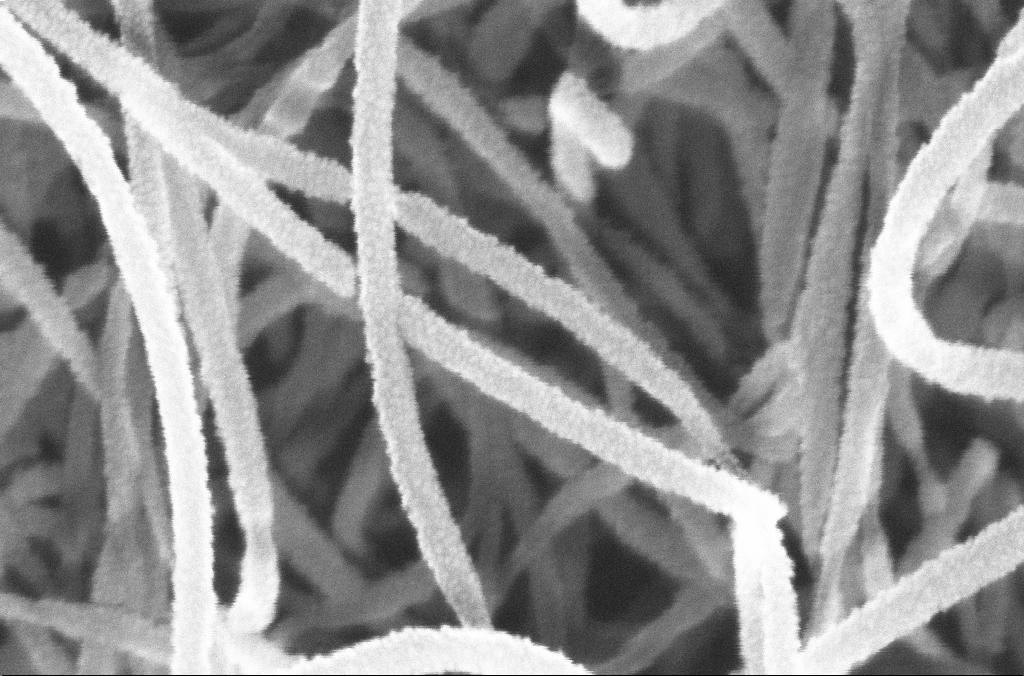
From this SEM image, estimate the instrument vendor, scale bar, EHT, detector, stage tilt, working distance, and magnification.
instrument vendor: Zeiss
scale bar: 100 nm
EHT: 20 kV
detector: InLens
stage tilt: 0°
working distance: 4.5 mm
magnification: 400 K X